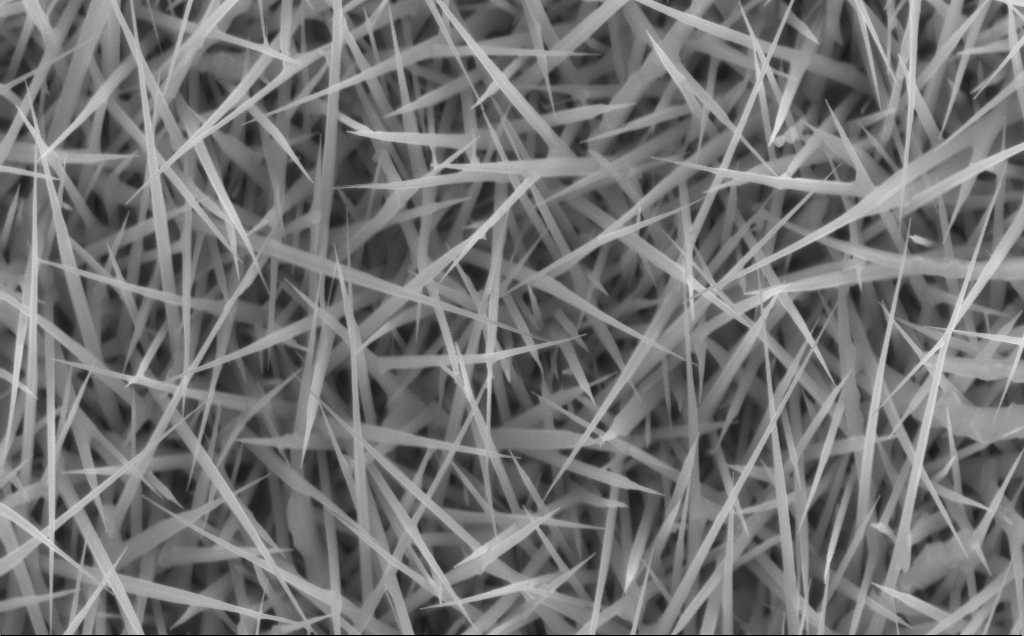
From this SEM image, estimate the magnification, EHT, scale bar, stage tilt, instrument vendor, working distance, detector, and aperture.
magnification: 40 K X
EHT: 10 kV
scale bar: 1000 nm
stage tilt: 0°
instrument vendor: Zeiss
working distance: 7 mm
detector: InLens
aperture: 30 µm